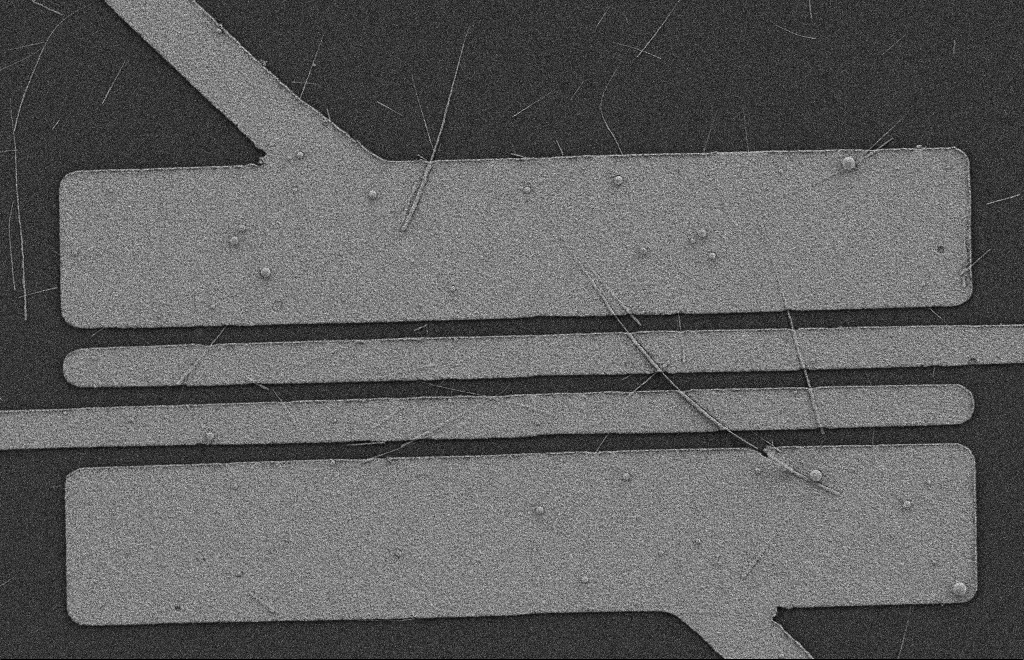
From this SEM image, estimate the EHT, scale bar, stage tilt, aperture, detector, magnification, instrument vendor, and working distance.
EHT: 2 kV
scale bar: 2000 nm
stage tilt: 0°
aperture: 20 µm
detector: SE2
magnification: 5.46 K X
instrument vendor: Zeiss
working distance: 9 mm